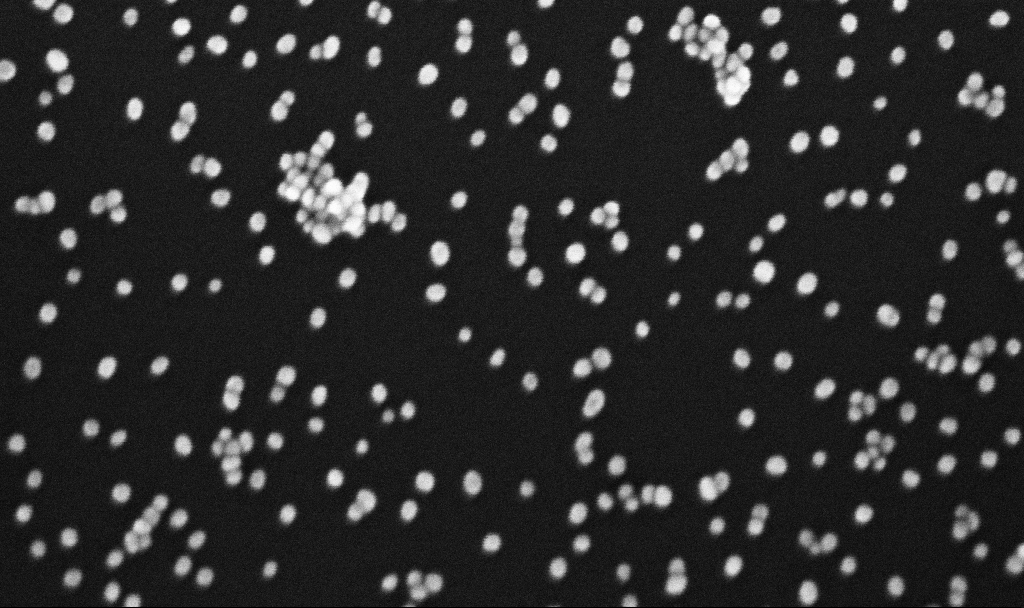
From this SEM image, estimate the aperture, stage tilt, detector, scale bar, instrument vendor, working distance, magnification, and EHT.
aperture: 30 µm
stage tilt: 0°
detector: InLens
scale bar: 200 nm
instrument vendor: Zeiss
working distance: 5.4 mm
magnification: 300 K X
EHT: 10 kV